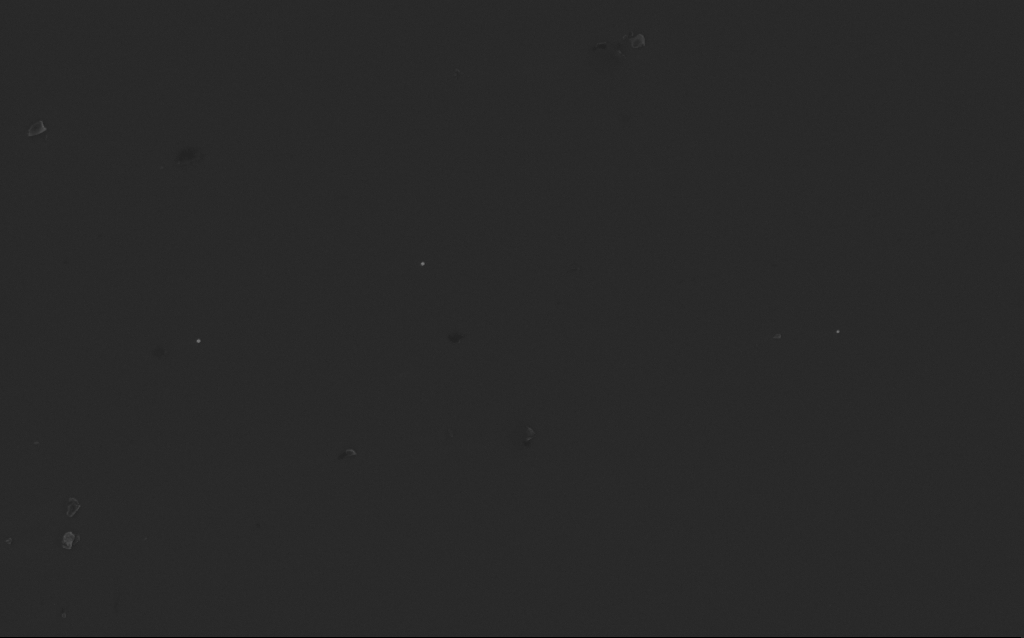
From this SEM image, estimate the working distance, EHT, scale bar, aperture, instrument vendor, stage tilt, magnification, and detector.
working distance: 3 mm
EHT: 5 kV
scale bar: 1000 nm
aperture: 30 µm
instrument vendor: Zeiss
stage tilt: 0°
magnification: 18.94 K X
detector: InLens